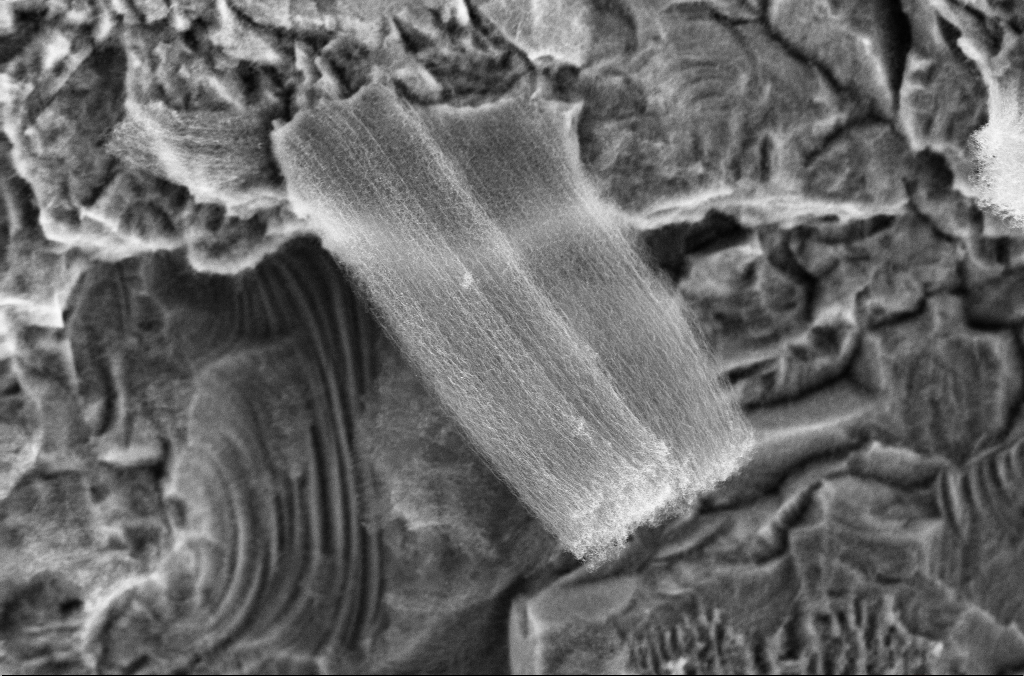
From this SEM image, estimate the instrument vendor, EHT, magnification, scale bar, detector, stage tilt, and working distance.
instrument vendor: Zeiss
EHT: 10 kV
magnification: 6.54 K X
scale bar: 2000 nm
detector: InLens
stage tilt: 0°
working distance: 4 mm